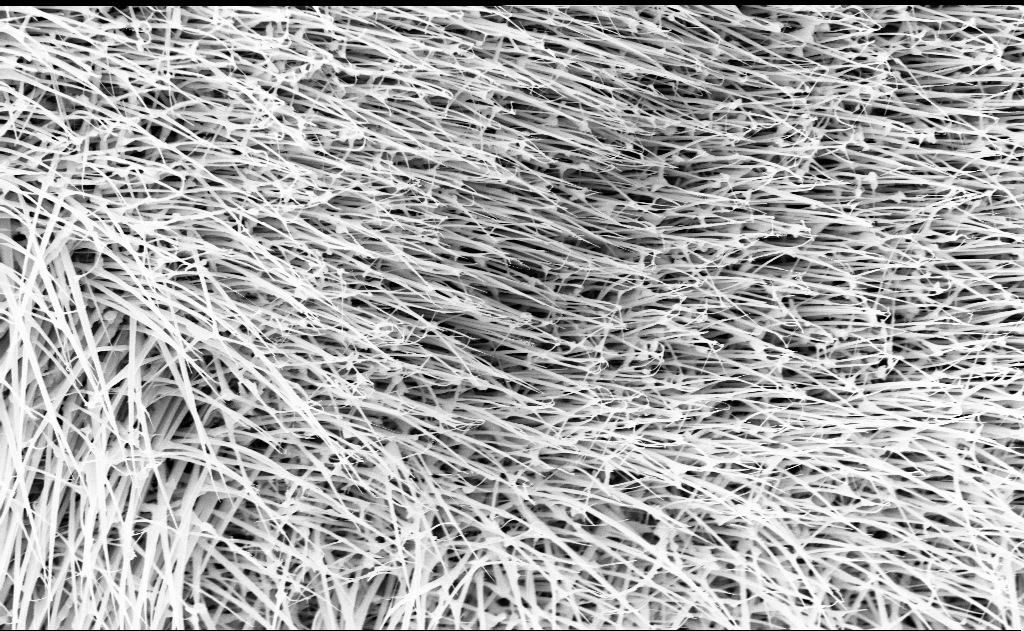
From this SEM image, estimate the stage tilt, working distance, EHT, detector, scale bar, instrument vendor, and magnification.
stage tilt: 0°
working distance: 13 mm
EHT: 10 kV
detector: InLens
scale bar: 1000 nm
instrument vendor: Zeiss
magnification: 20 K X